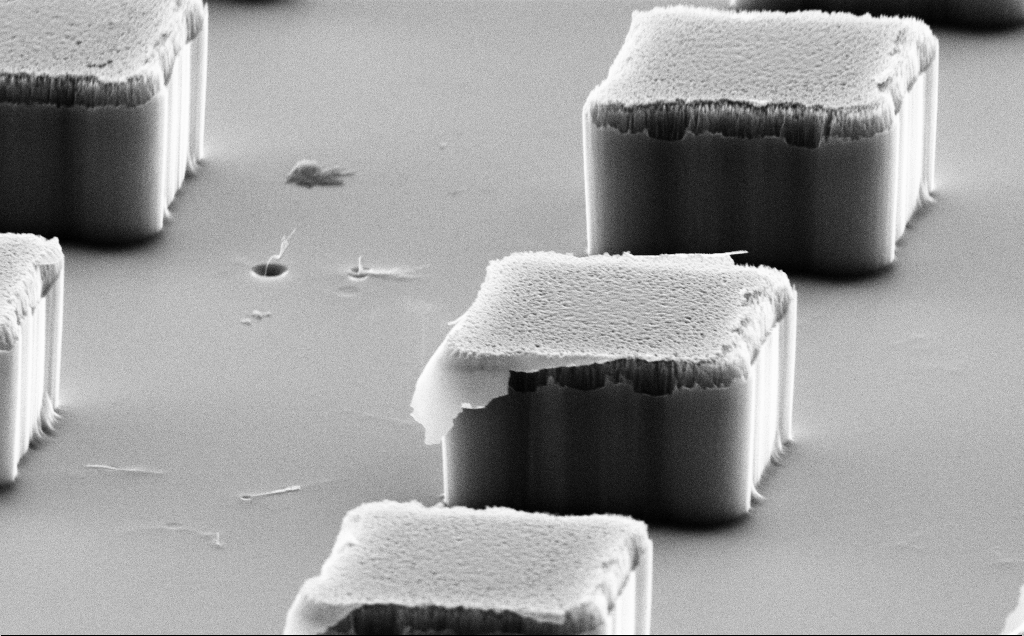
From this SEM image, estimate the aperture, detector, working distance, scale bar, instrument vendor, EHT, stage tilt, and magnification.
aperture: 30 µm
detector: SE2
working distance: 18 mm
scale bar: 1000 nm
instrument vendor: Zeiss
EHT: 10 kV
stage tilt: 70°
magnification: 13.59 K X